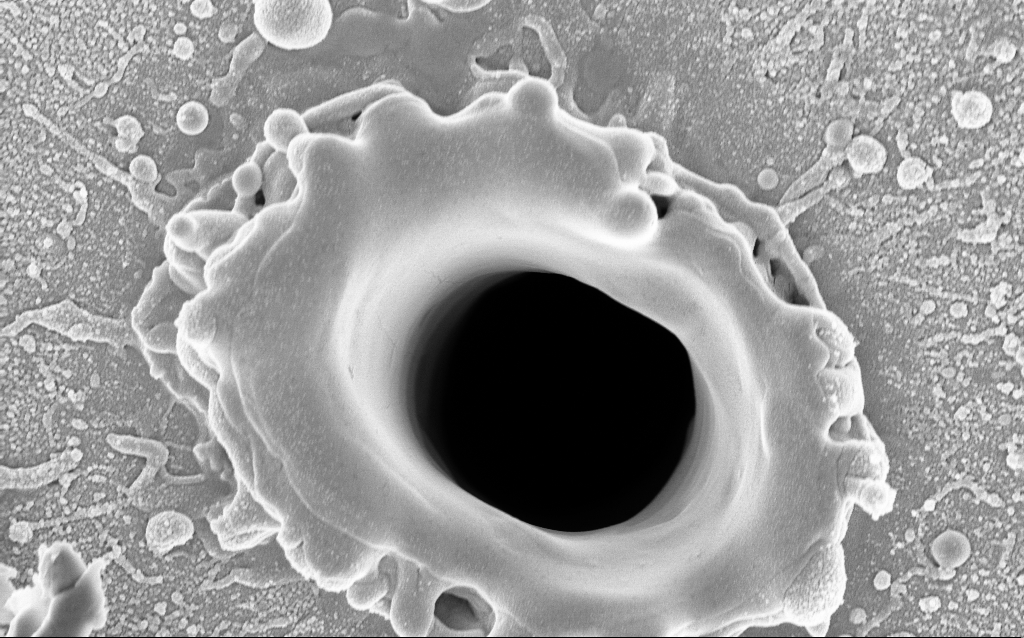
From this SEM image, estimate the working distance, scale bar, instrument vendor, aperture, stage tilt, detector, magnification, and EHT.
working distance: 4.3 mm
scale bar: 2000 nm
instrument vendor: Zeiss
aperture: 30 µm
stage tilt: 0°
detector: InLens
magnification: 36.18 K X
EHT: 5 kV